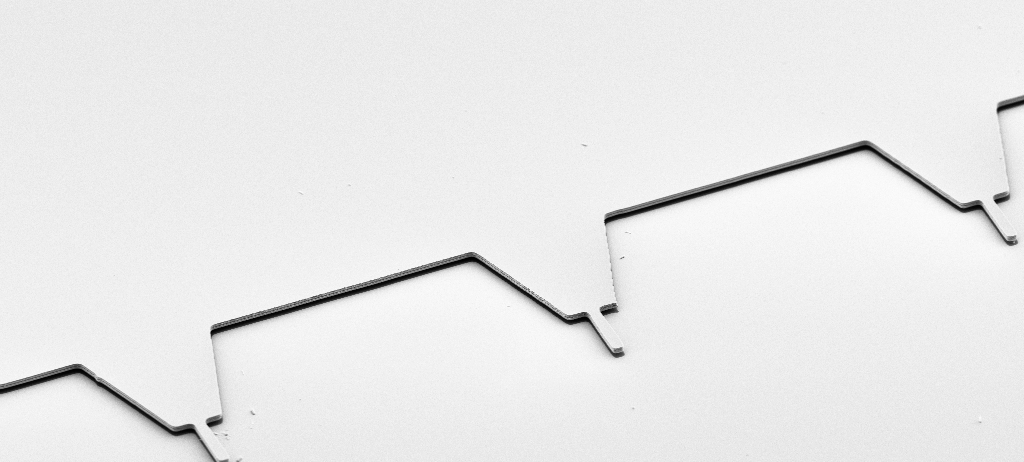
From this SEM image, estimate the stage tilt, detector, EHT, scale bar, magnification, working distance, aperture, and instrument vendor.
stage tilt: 50°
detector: SE2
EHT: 5 kV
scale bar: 20000 nm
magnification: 0.989 K X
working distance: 10 mm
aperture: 30 µm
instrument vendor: Zeiss